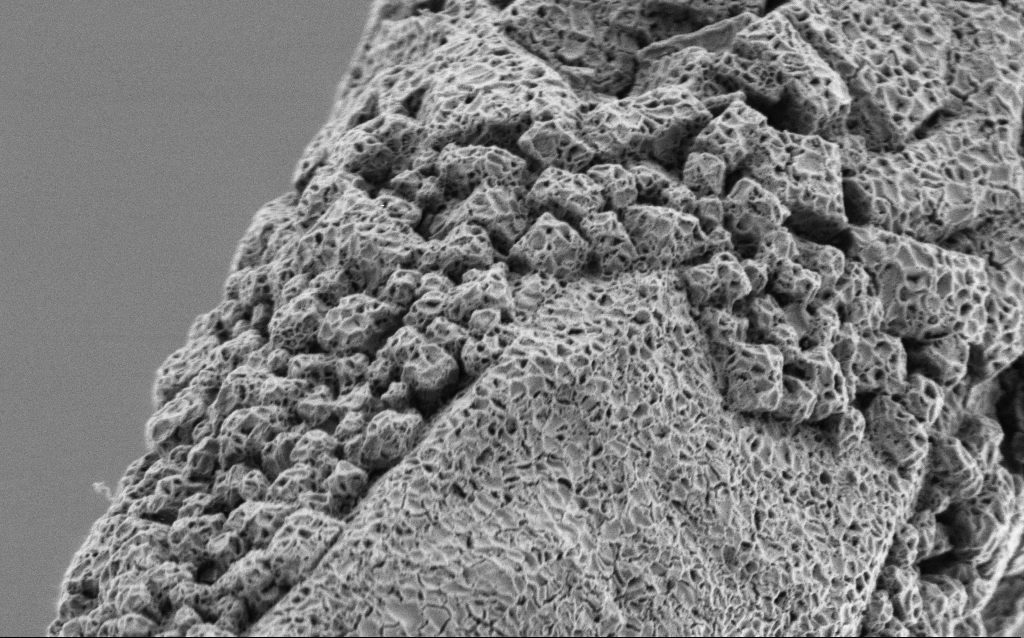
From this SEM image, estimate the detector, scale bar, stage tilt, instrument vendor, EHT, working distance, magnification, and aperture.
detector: SE2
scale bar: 2000 nm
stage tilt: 45°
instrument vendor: Zeiss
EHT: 1 kV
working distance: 6.8 mm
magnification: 25 K X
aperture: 30 µm